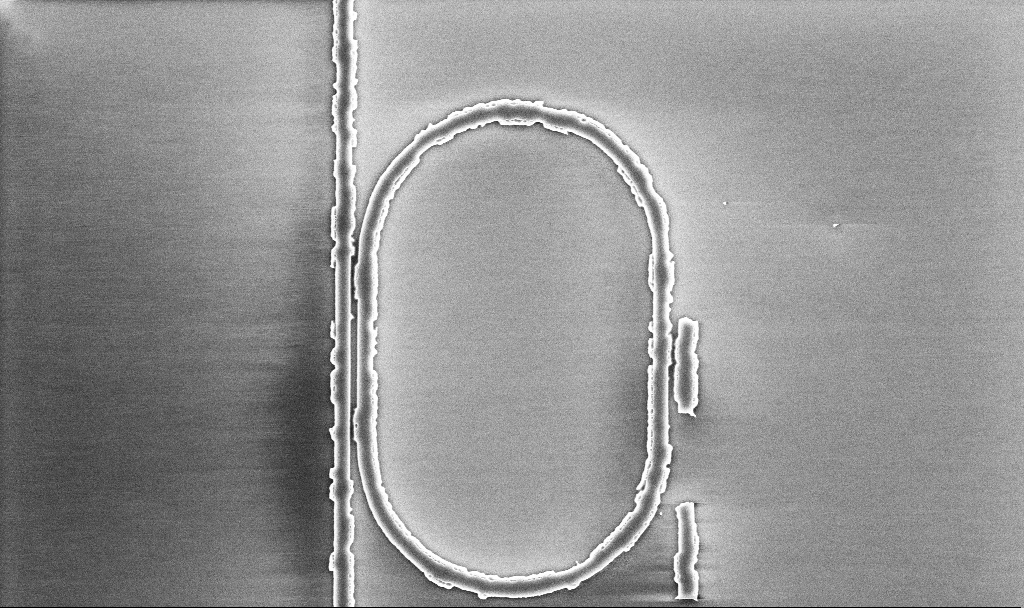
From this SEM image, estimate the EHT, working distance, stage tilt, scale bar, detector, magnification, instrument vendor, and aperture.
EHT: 5 kV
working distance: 10.1 mm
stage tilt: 0°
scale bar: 2000 nm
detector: InLens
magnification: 11.5 K X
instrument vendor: Zeiss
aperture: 30 µm